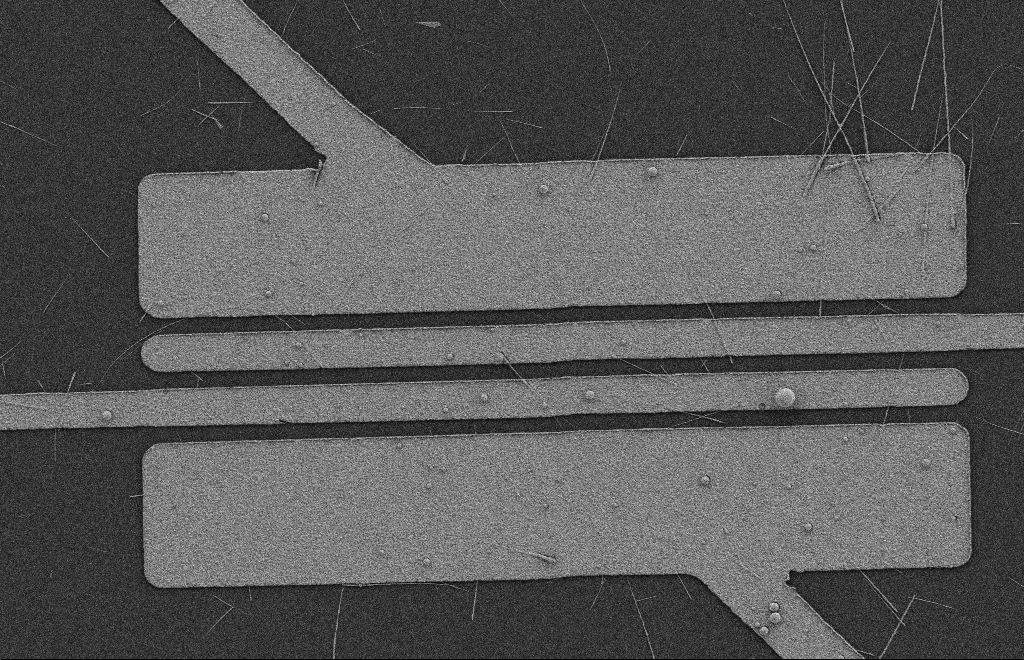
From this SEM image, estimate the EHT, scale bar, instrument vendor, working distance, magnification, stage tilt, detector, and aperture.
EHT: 2 kV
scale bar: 2000 nm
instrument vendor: Zeiss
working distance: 9 mm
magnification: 4.97 K X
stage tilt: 0°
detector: SE2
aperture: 20 µm